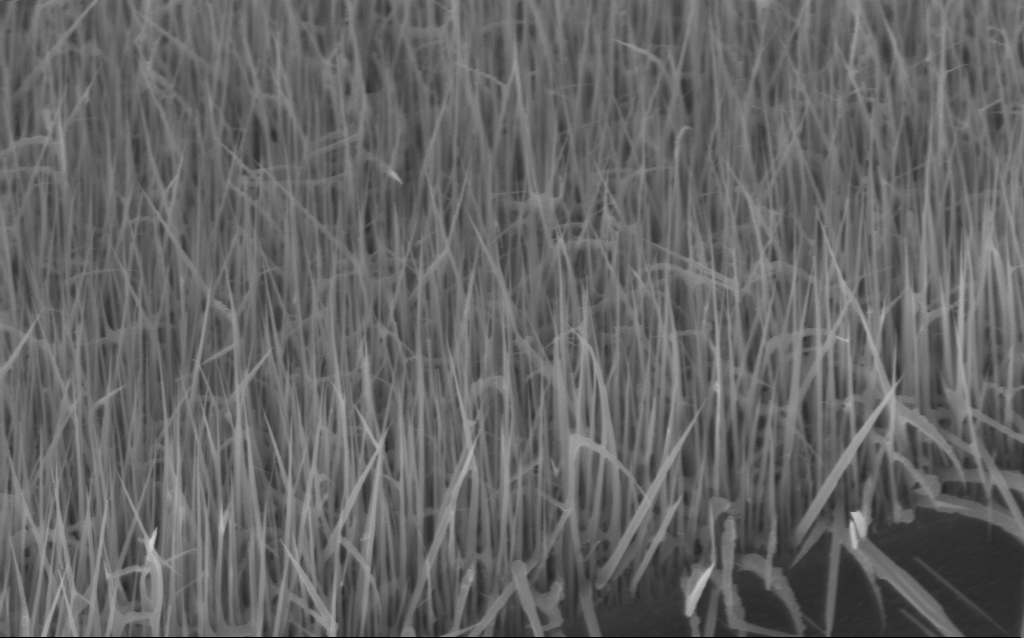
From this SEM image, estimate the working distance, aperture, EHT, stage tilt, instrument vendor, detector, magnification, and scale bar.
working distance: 6 mm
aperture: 30 µm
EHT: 10 kV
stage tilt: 45°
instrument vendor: Zeiss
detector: InLens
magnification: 41.12 K X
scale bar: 1000 nm